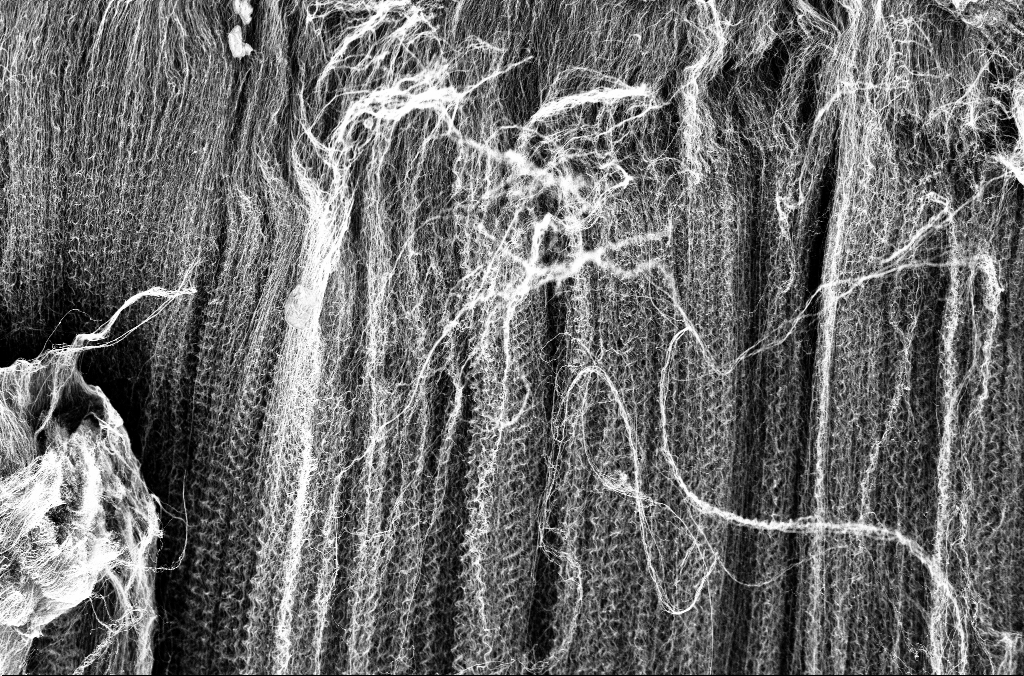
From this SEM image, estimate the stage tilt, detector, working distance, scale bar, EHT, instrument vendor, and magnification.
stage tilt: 45°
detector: InLens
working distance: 3.3 mm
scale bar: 10000 nm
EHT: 3 kV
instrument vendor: Zeiss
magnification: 5 K X